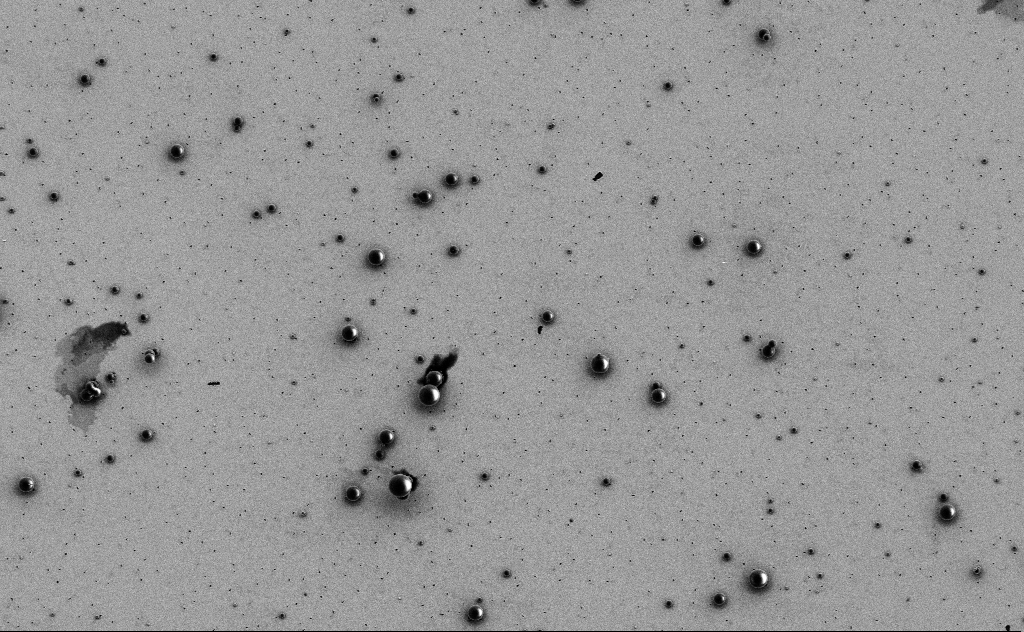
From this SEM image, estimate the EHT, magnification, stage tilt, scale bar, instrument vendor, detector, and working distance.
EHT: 3 kV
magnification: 3.23 K X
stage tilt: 0°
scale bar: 10000 nm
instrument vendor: Zeiss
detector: SE2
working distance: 11 mm